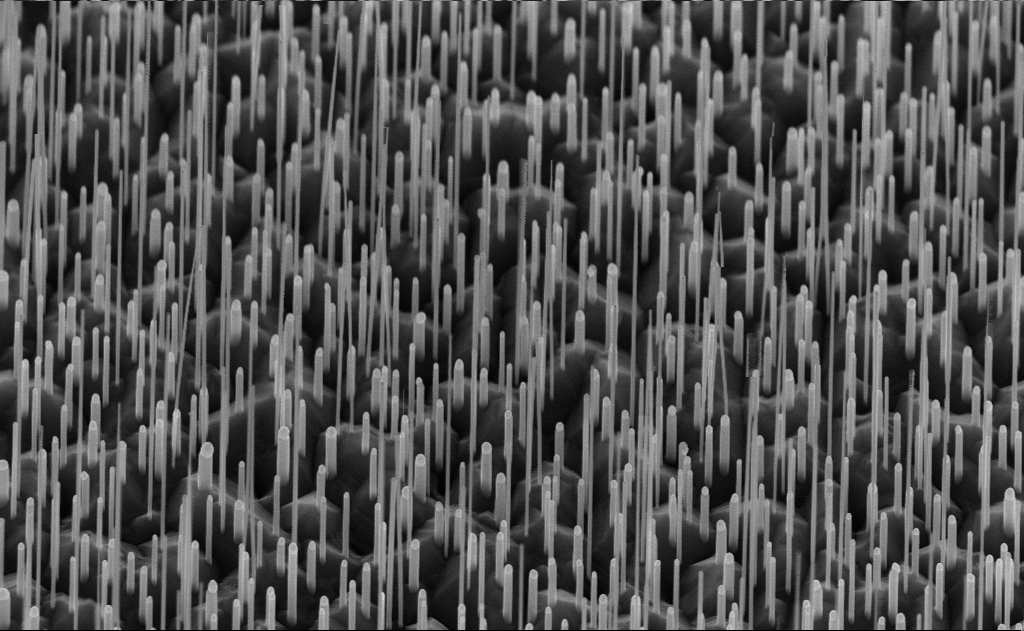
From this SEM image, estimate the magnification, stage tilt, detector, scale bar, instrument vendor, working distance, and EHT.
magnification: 40.14 K X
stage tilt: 45°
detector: InLens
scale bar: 1000 nm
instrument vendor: Zeiss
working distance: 6 mm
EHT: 10 kV